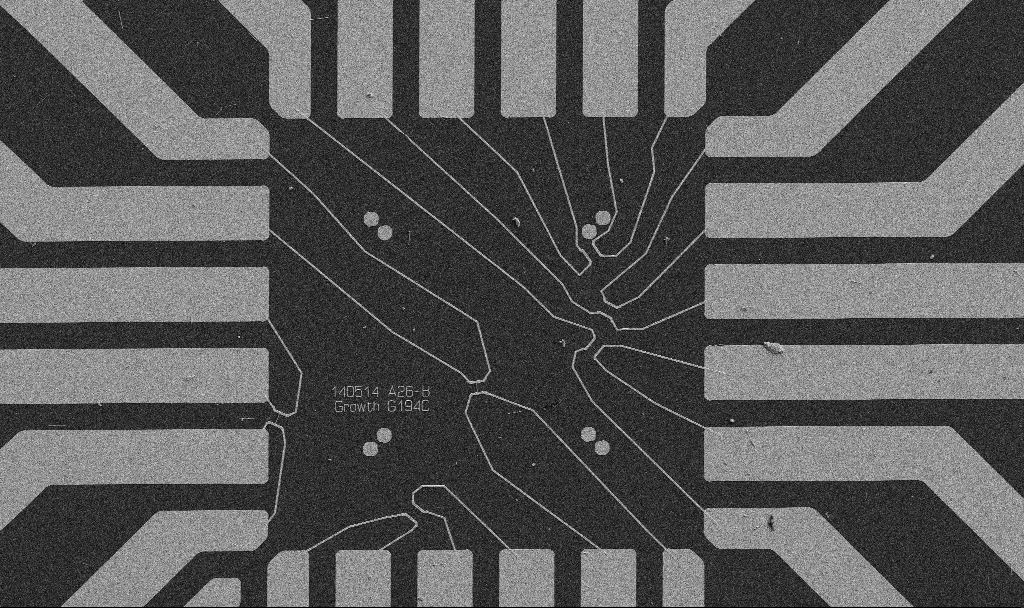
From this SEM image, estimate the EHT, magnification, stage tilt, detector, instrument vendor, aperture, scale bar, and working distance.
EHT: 5 kV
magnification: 1 K X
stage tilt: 0°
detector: SE2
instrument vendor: Zeiss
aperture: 30 µm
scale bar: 20000 nm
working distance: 10.7 mm